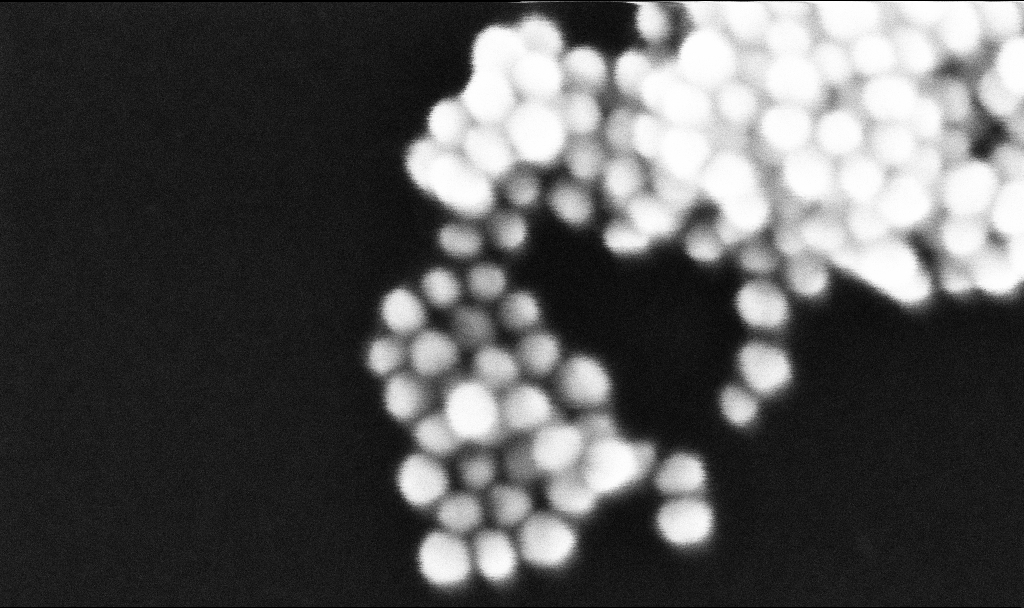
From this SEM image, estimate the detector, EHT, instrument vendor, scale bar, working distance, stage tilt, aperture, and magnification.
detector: InLens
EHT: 10 kV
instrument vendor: Zeiss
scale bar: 20 nm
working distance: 3.2 mm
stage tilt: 0°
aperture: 30 µm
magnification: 857.1 K X